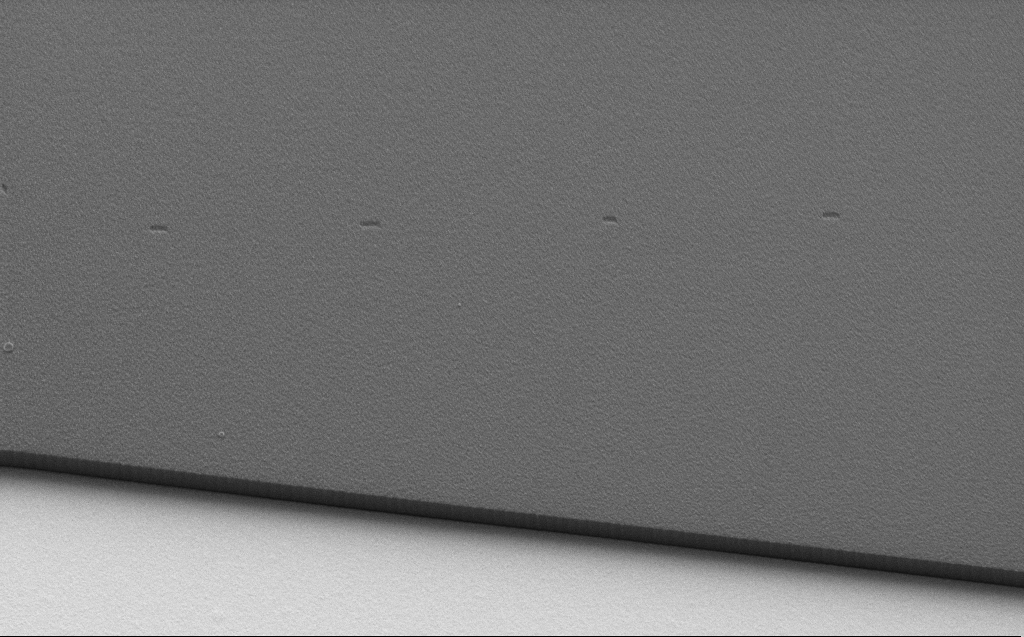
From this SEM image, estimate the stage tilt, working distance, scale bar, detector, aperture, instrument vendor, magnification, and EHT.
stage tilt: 30°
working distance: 6 mm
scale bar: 10000 nm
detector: SE2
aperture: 30 µm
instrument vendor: Zeiss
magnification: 5.93 K X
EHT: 1.1 kV